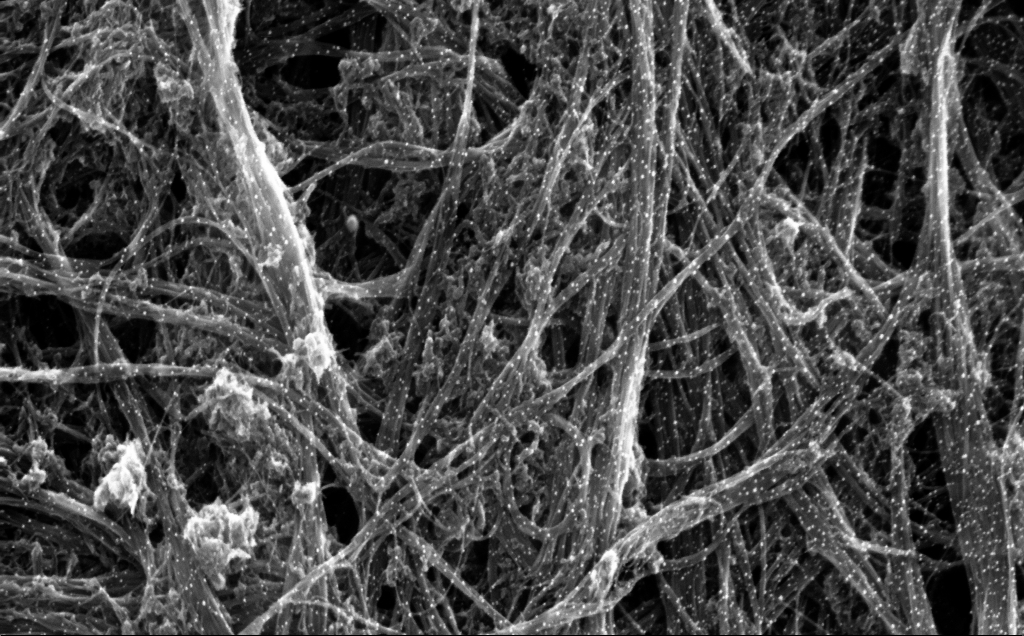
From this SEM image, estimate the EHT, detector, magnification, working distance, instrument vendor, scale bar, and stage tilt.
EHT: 5 kV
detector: InLens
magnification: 136.34 K X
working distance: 4 mm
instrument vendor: Zeiss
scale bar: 200 nm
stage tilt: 0°